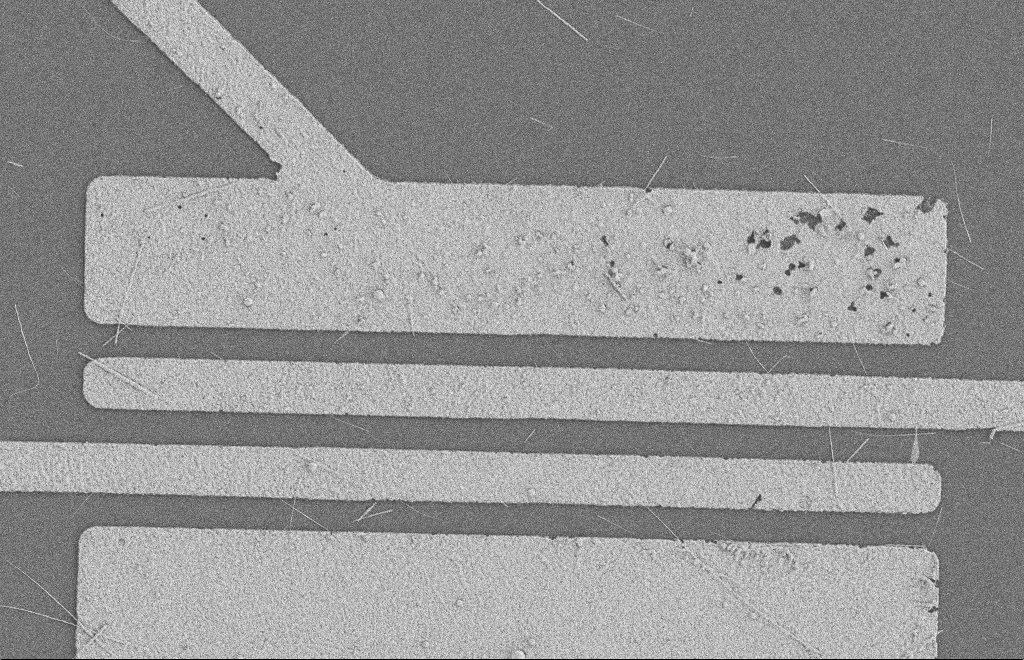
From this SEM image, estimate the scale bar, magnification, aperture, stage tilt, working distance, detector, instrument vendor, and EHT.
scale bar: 2000 nm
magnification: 5.17 K X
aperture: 20 µm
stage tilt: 0°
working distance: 8 mm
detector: SE2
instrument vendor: Zeiss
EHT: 2 kV